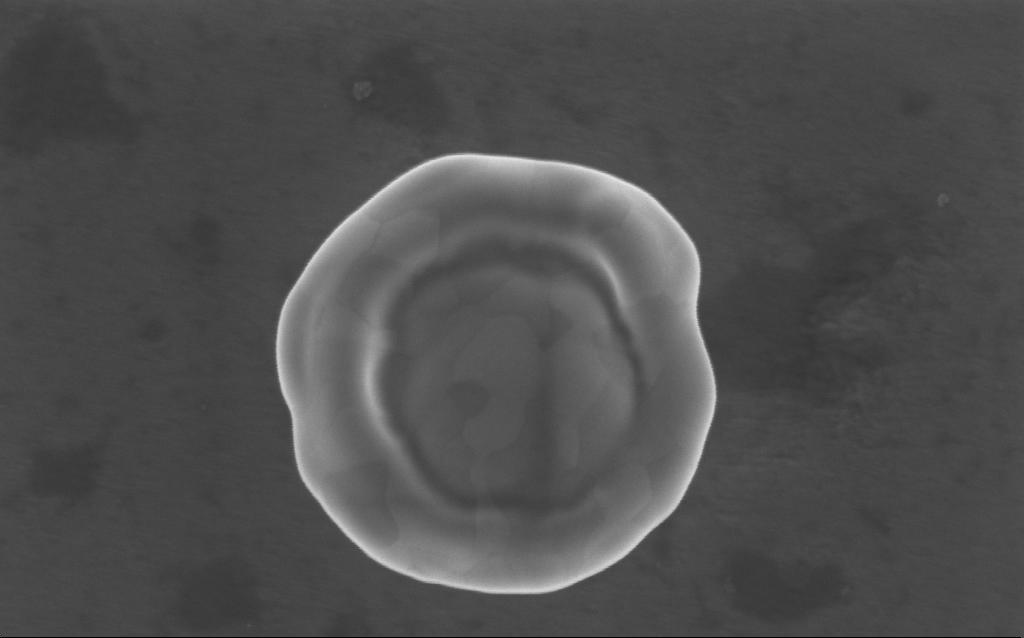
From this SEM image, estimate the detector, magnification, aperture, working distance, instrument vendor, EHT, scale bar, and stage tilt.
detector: InLens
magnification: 120.92 K X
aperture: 30 µm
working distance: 4 mm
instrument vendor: Zeiss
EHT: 5 kV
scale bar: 200 nm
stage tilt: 0°